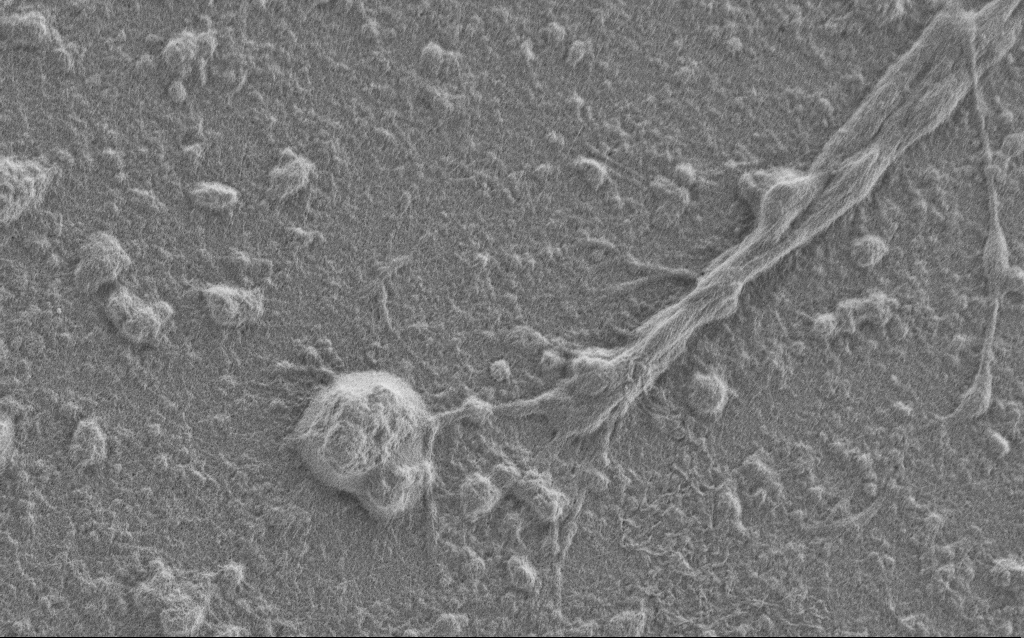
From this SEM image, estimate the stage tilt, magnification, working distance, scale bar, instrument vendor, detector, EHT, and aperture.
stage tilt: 0°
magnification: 7.5 K X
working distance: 6 mm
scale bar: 2000 nm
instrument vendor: Zeiss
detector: SE2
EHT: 1 kV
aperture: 30 µm